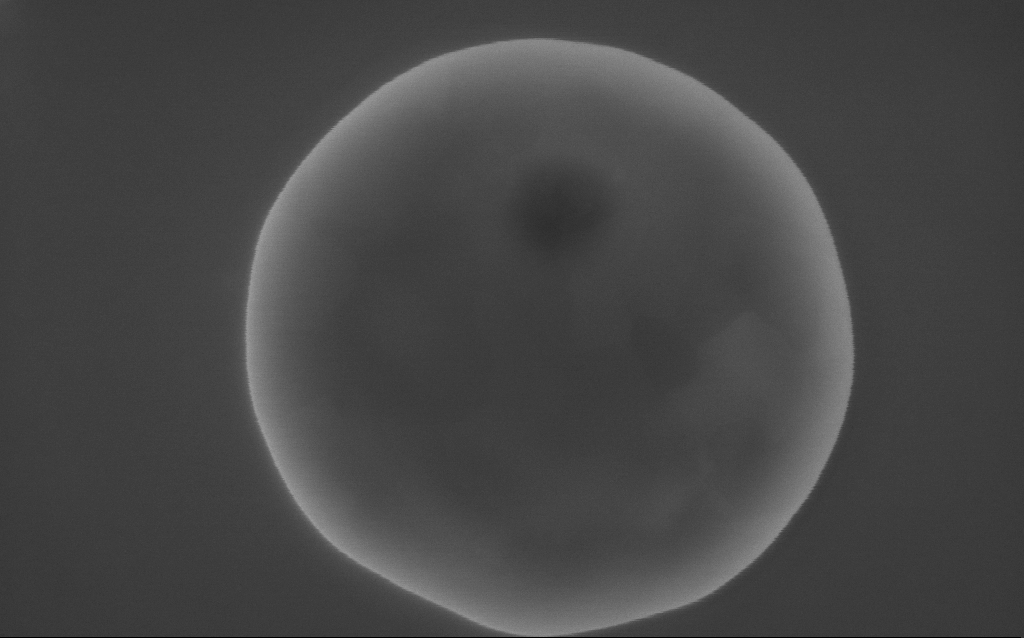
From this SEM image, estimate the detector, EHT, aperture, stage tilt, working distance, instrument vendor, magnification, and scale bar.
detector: InLens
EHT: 10 kV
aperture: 30 µm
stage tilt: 0°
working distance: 2 mm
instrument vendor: Zeiss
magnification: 175 K X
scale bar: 200 nm